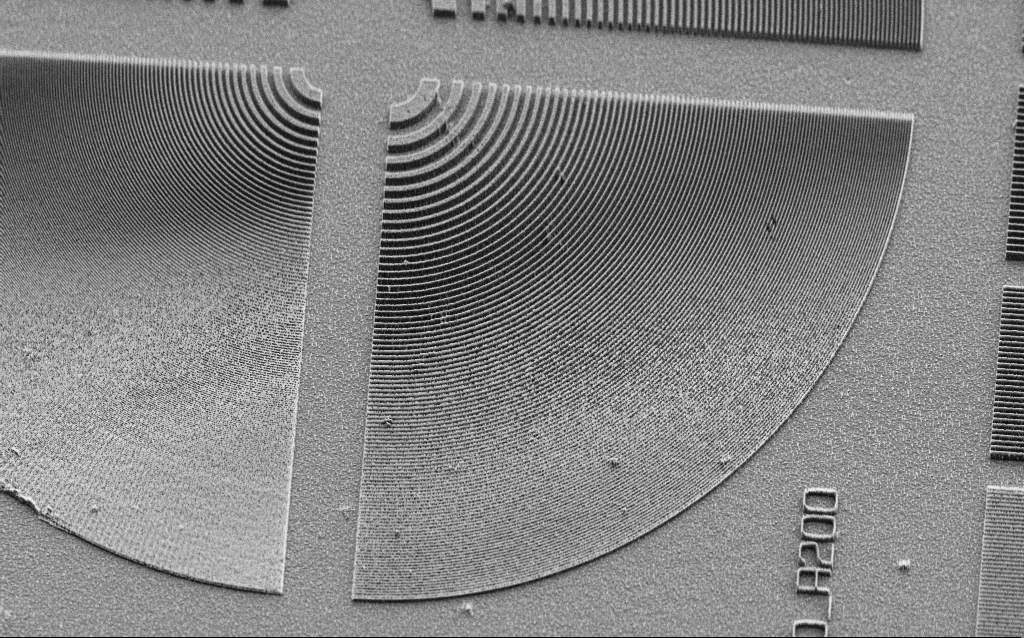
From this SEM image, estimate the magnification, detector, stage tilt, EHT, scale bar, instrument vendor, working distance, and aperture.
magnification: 2.56 K X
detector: SE2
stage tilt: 45°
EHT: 3 kV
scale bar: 10000 nm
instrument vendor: Zeiss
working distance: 8 mm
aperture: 30 µm